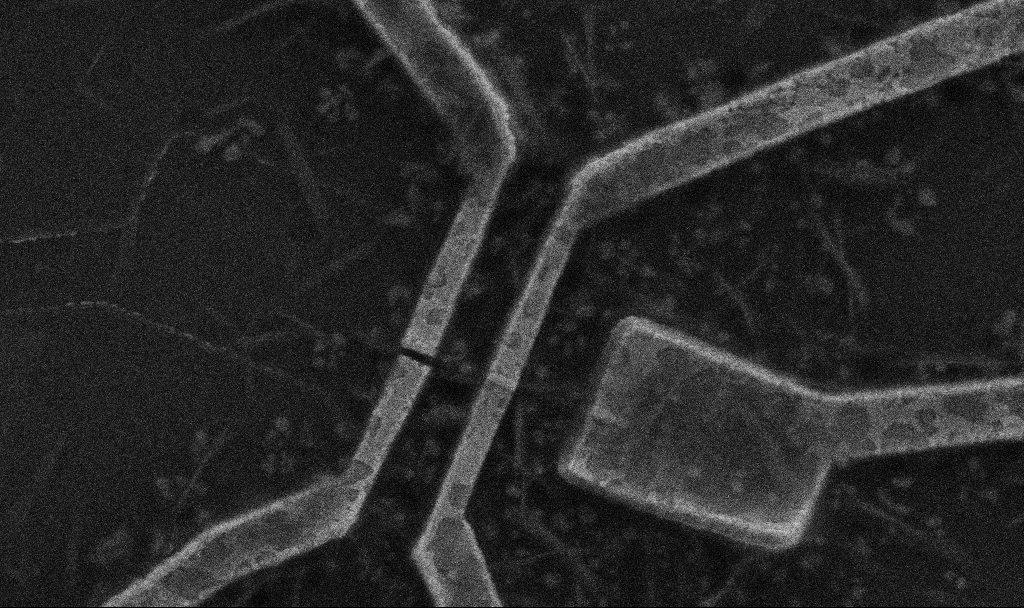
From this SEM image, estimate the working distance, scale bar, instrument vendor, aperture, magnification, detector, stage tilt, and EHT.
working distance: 9.7 mm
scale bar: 2000 nm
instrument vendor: Zeiss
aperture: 30 µm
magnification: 30 K X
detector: SE2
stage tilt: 0°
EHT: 5 kV